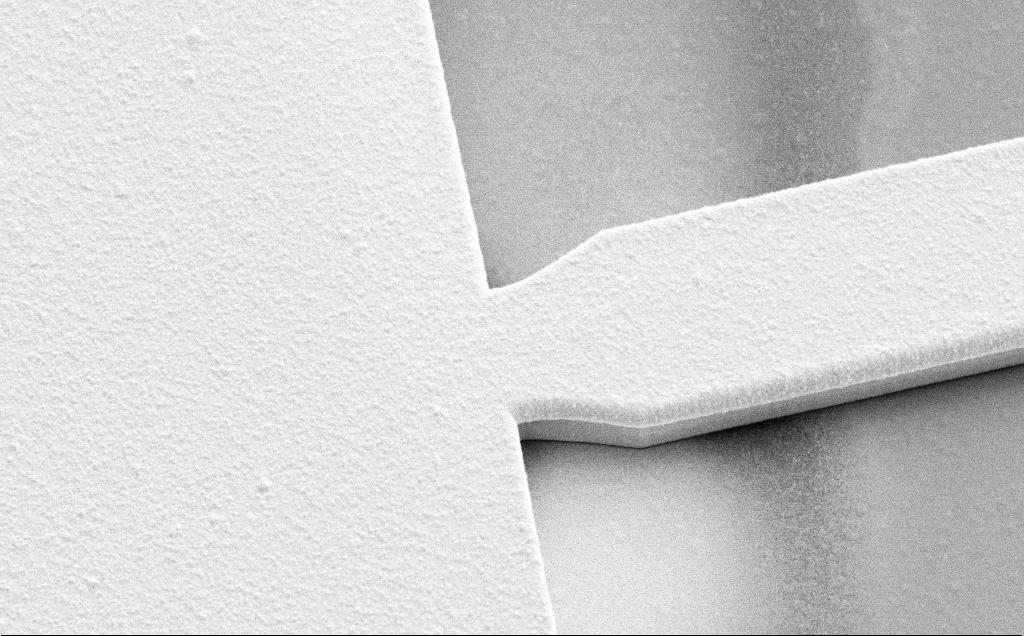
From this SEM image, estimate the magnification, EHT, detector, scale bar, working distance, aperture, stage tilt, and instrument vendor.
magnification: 6.45 K X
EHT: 10 kV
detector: SE2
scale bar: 10000 nm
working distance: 12 mm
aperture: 30 µm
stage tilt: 45°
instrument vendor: Zeiss